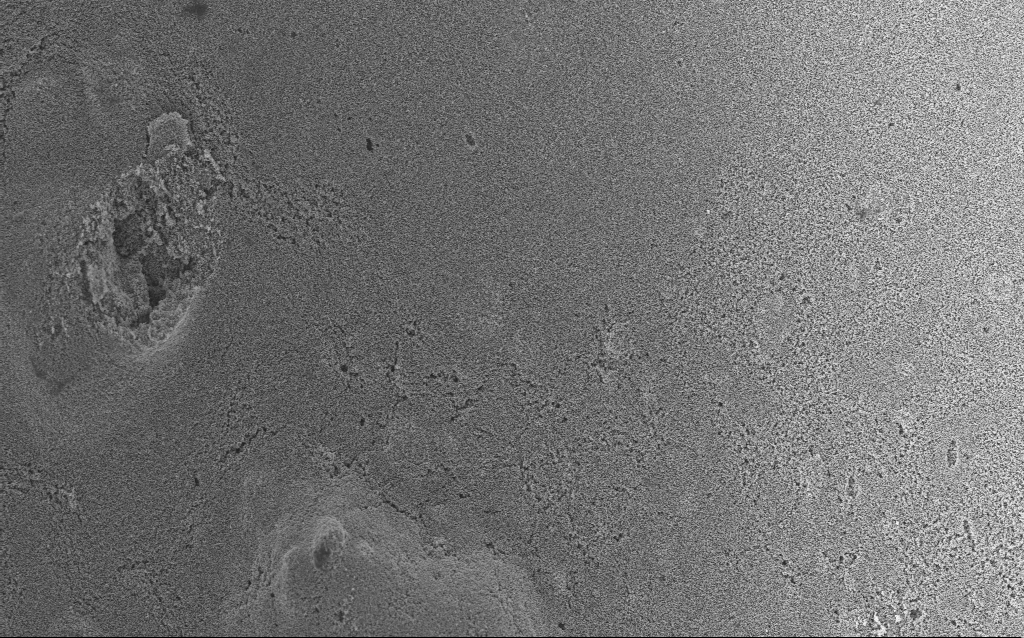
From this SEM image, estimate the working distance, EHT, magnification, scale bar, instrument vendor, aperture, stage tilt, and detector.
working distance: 2.7 mm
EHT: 3 kV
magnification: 5.83 K X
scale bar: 10000 nm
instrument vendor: Zeiss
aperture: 30 µm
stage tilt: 0°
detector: InLens